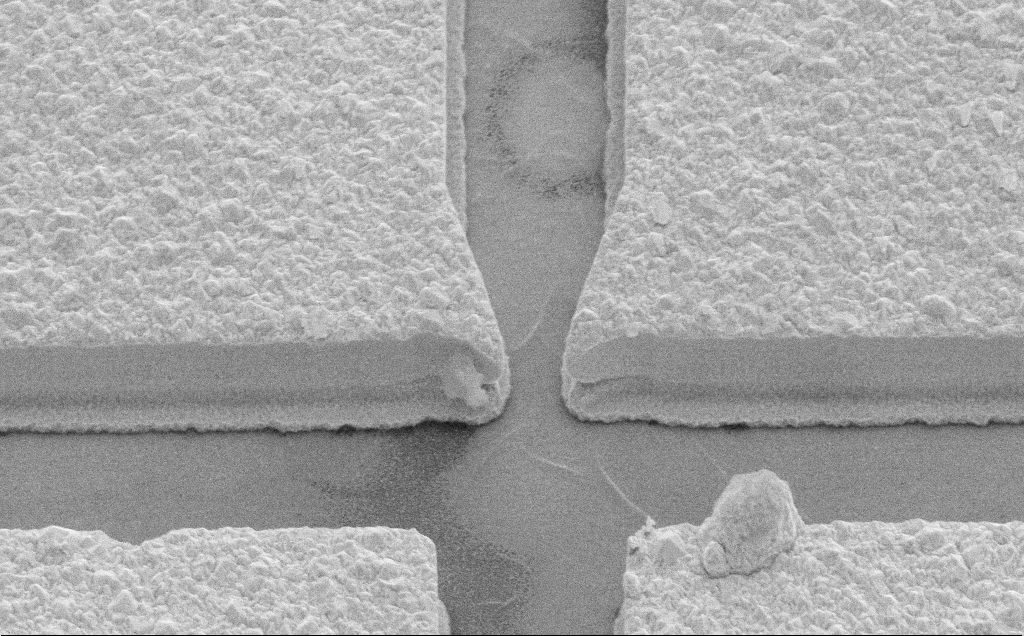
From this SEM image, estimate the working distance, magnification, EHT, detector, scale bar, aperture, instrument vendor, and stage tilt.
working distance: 11 mm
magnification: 6.96 K X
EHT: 10 kV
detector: SE2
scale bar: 10000 nm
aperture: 30 µm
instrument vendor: Zeiss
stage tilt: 45°